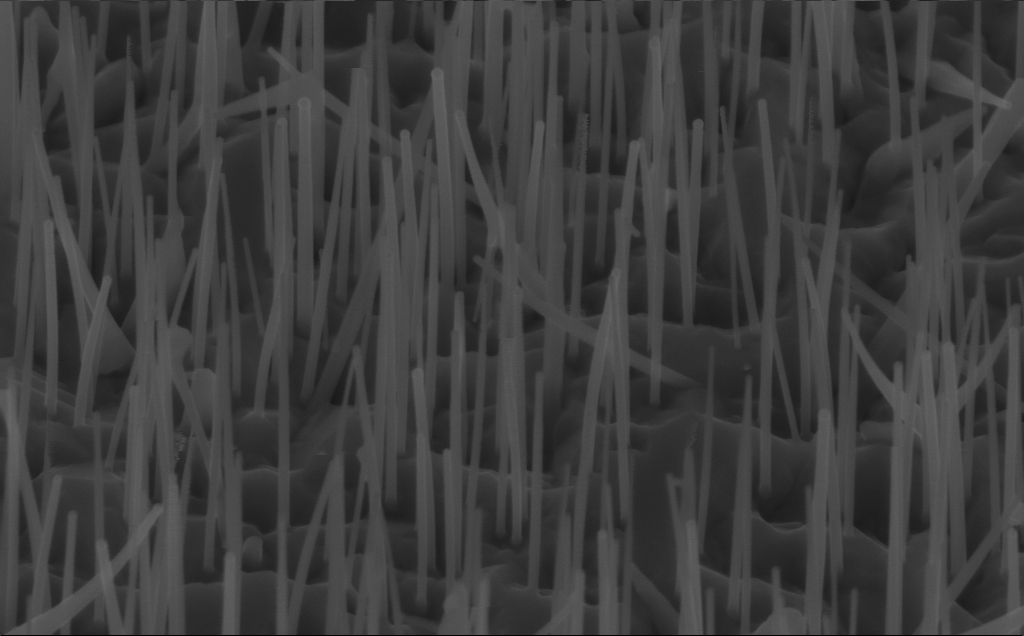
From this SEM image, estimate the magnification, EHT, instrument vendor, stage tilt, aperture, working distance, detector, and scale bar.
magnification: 80 K X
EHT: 10 kV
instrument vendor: Zeiss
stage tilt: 45°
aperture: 30 µm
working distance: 5 mm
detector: InLens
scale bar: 200 nm